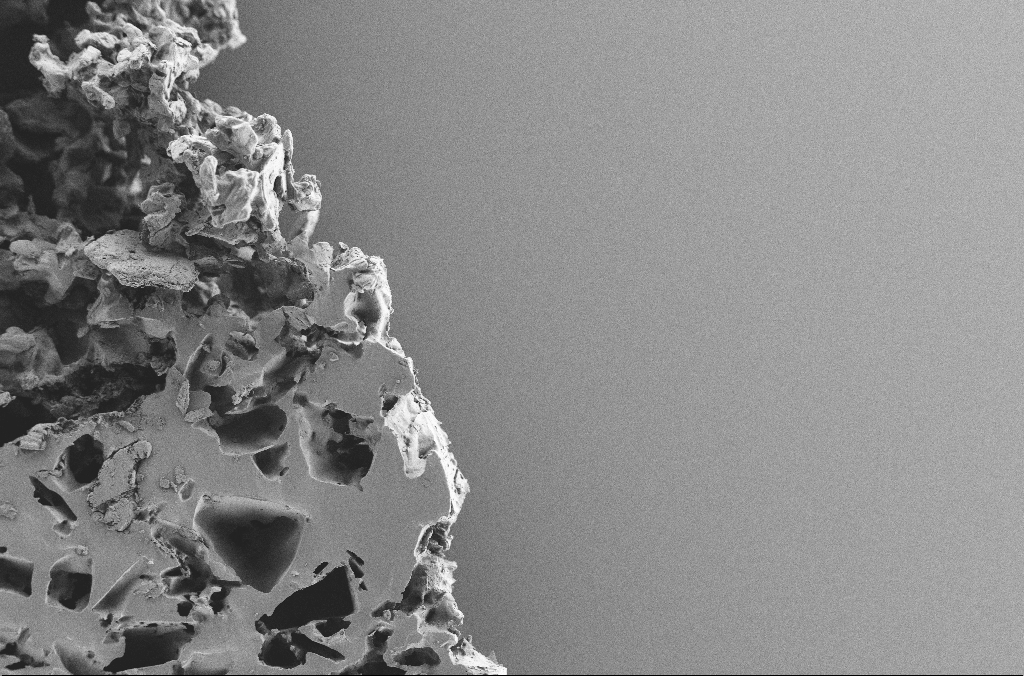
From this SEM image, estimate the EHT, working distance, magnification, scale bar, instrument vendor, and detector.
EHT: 2 kV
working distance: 3.1 mm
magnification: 0.25 K X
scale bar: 100000 nm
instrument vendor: Zeiss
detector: SE2